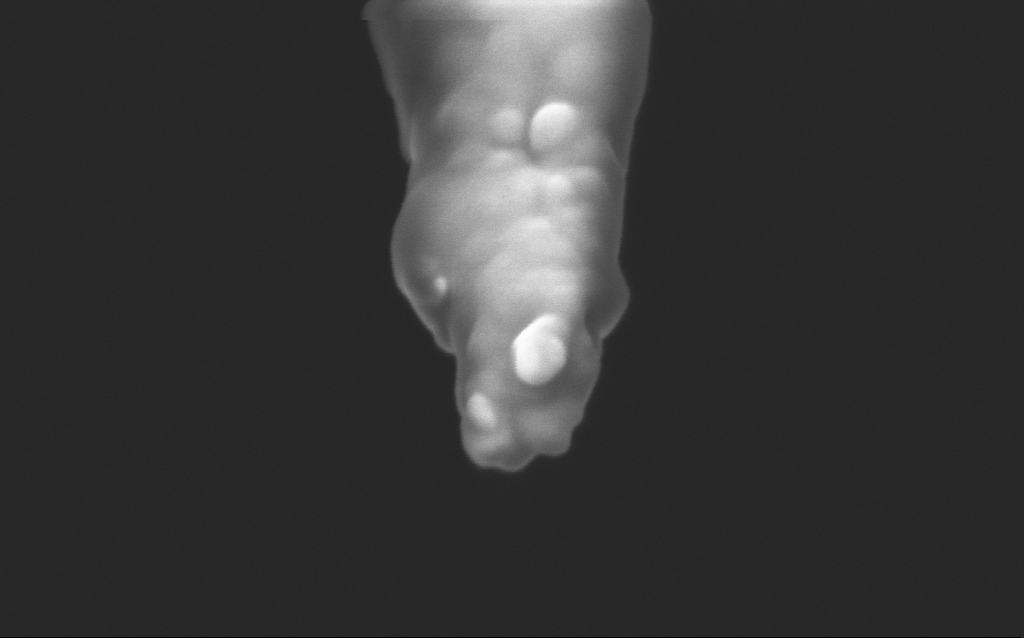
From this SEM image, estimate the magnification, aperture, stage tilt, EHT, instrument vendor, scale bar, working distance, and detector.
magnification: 250 K X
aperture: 30 µm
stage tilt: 45°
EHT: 2.5 kV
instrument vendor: Zeiss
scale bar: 200 nm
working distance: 5 mm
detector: InLens